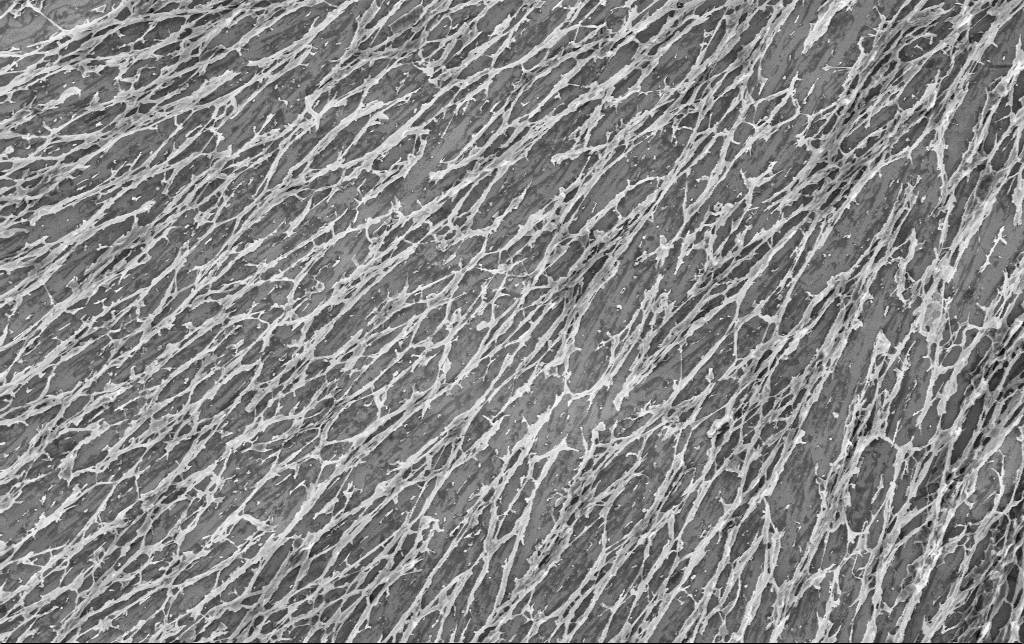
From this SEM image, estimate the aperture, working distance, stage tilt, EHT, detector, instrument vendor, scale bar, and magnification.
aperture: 30 µm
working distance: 3.4 mm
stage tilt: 0°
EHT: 3 kV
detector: InLens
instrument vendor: Zeiss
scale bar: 10000 nm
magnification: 5.2 K X